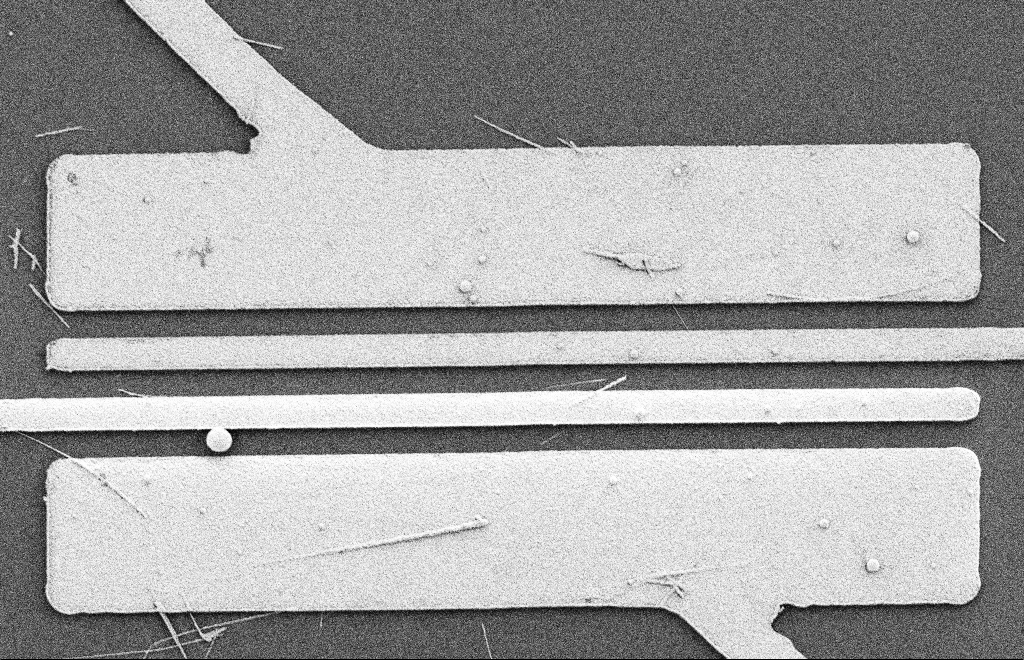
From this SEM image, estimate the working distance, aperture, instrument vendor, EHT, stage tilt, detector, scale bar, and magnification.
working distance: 10 mm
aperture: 20 µm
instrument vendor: Zeiss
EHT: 2 kV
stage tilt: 0°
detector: SE2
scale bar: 2000 nm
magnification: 5.61 K X